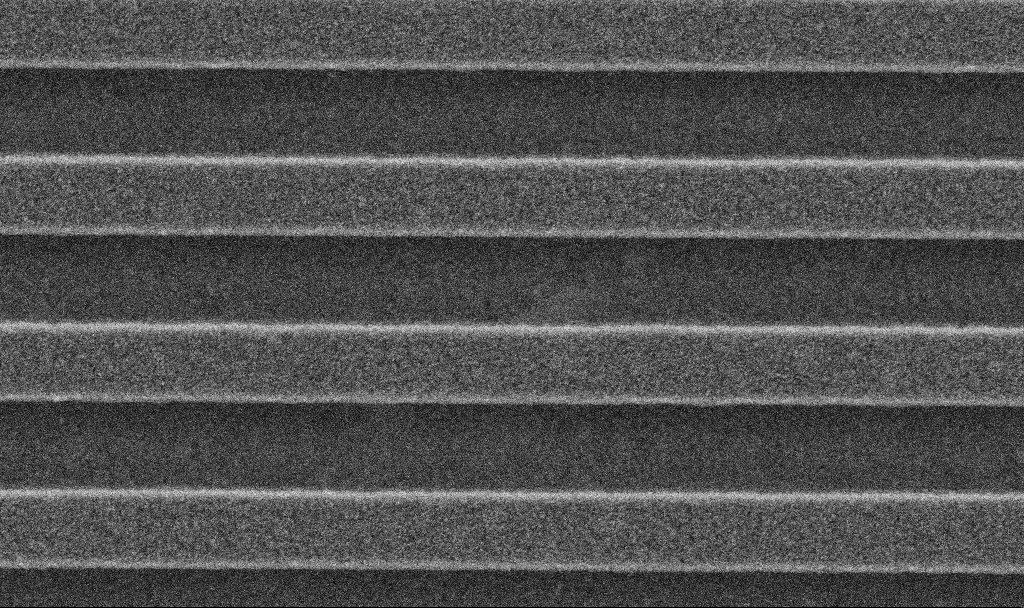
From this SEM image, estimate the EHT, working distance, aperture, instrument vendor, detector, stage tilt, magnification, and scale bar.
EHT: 5 kV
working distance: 2.9 mm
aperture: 30 µm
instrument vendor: Zeiss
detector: SE2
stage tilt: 0°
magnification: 76.83 K X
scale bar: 200 nm